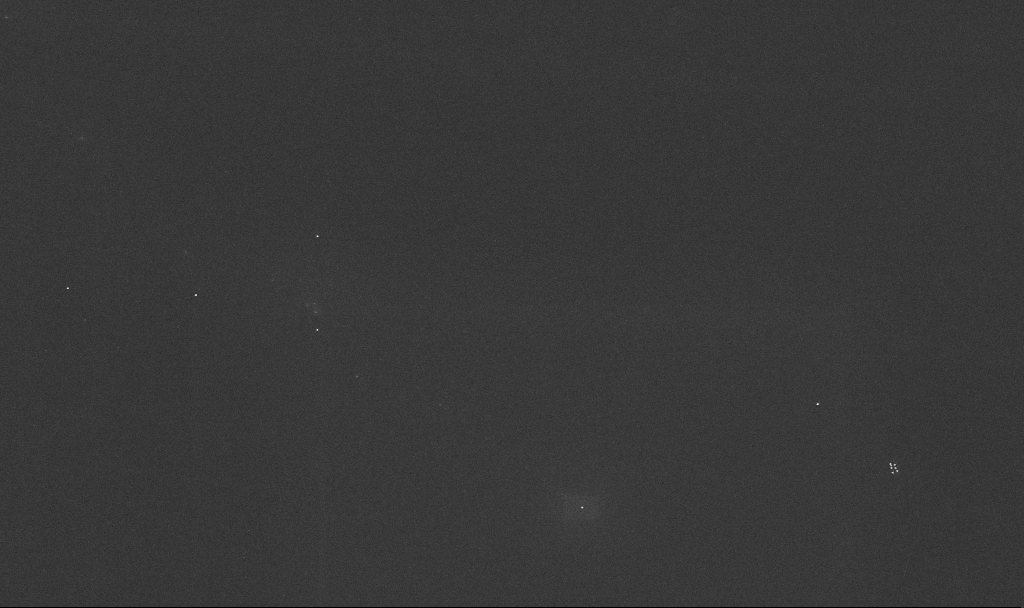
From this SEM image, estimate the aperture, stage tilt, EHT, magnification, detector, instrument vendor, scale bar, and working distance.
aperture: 30 µm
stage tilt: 0°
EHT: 10 kV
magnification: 31.12 K X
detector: InLens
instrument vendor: Zeiss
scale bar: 1000 nm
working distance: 3.2 mm